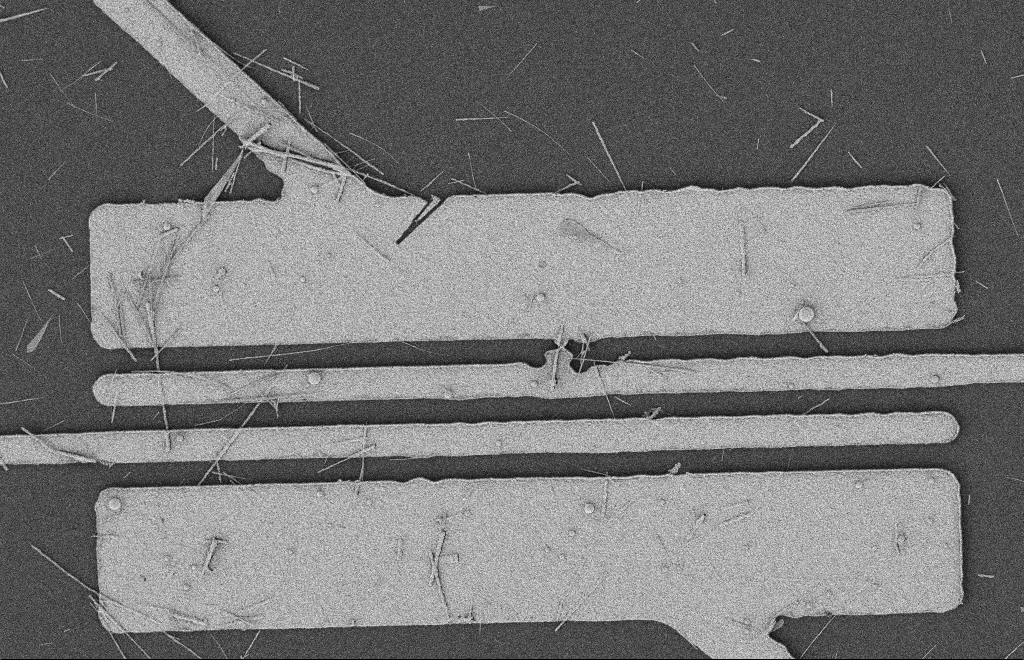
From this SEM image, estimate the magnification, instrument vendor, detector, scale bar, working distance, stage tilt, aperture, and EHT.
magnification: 5.18 K X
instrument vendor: Zeiss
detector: SE2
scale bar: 2000 nm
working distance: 12 mm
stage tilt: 0°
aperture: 20 µm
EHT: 2 kV